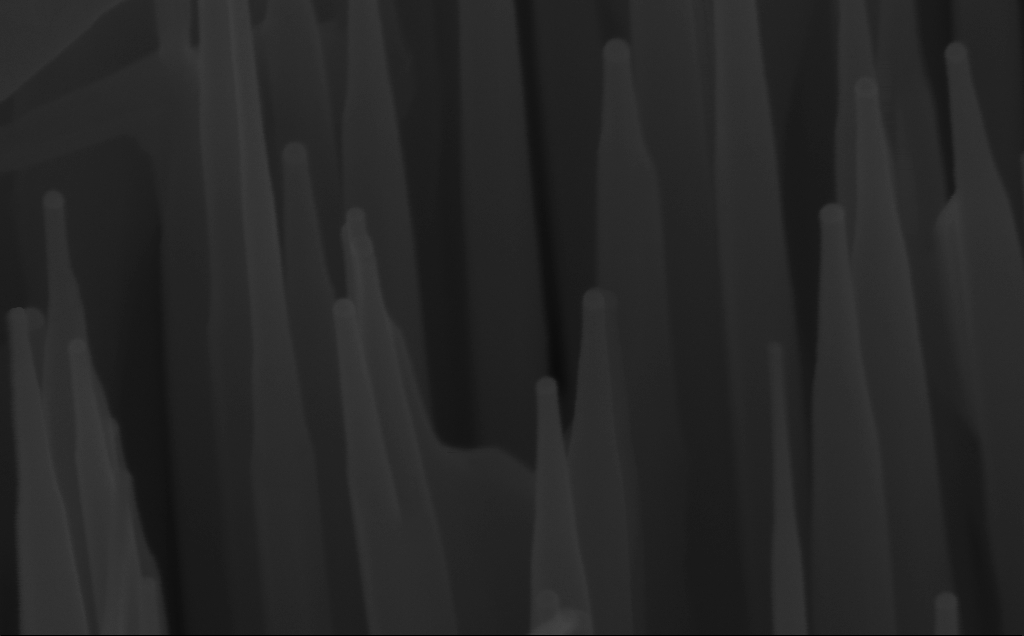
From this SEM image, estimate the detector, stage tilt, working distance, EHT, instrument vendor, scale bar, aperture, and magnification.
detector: InLens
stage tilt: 45°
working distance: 6 mm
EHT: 10 kV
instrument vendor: Zeiss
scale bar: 100 nm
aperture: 30 µm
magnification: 300 K X